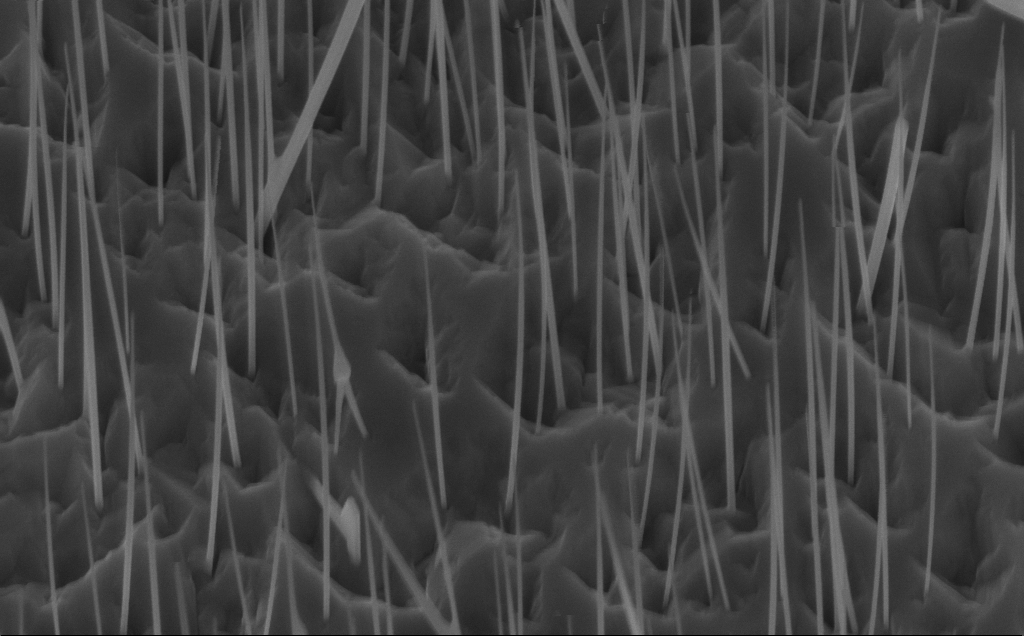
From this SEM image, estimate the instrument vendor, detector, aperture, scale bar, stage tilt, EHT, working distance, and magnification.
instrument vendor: Zeiss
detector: InLens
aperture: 30 µm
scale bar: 1000 nm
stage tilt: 45°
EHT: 10 kV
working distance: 7 mm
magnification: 49.22 K X